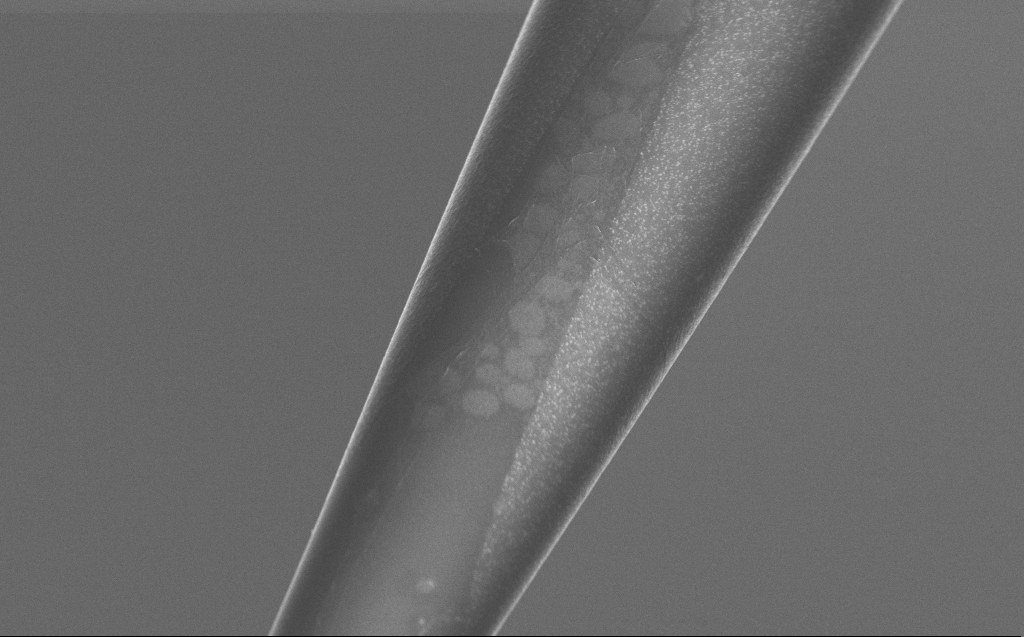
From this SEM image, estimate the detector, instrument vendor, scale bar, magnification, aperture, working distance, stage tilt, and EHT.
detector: InLens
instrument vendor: Zeiss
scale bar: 2000 nm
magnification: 10 K X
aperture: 30 µm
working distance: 6 mm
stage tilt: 45°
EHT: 5 kV